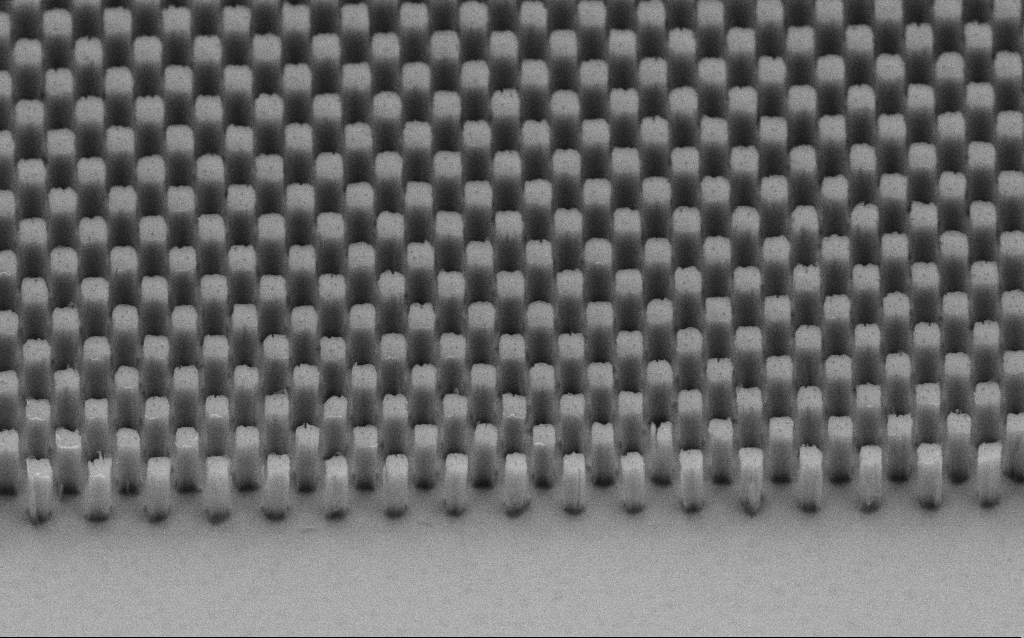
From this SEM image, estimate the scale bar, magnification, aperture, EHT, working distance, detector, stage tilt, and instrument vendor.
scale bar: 1000 nm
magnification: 21.68 K X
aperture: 30 µm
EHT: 5 kV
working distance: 7 mm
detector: SE2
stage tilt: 45°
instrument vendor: Zeiss